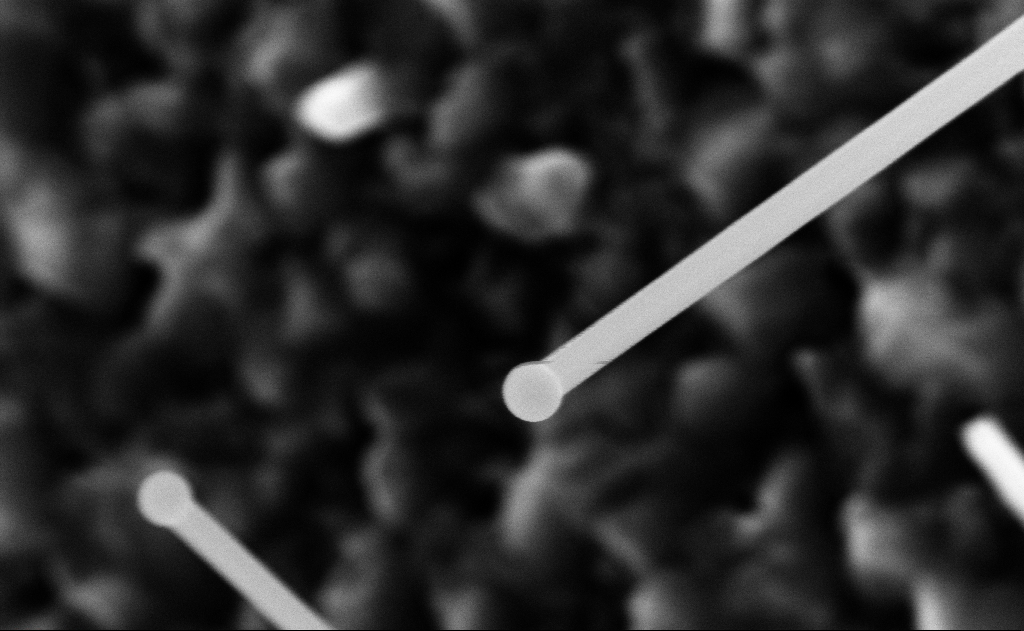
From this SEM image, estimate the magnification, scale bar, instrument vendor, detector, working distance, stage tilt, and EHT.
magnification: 150 K X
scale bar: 200 nm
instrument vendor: Zeiss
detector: InLens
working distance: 9 mm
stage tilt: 0°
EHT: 10 kV